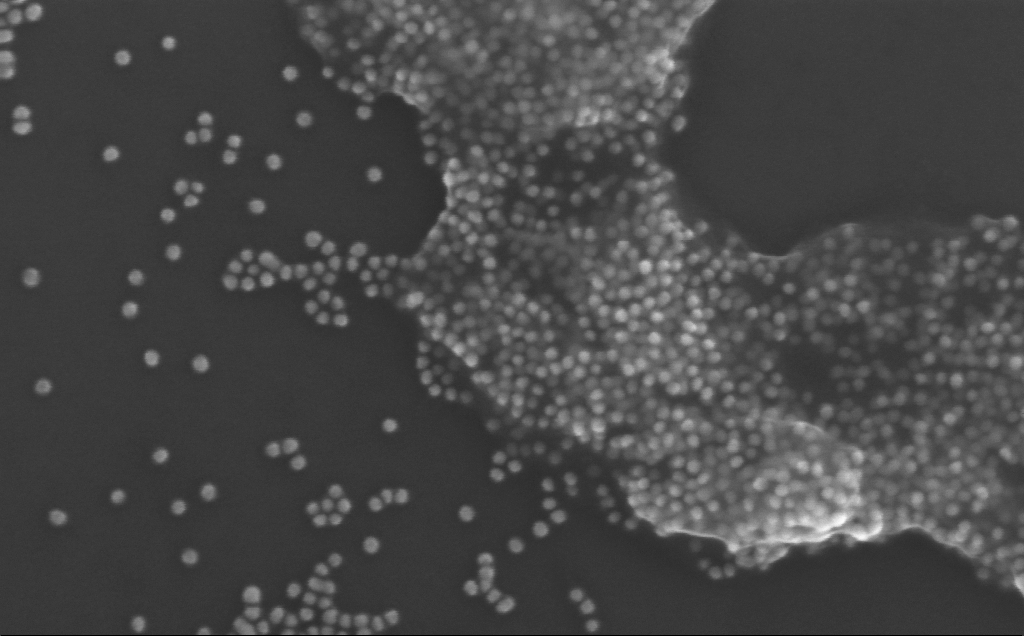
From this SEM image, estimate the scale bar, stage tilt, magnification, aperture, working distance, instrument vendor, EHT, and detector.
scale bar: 200 nm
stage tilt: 0°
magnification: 320.46 K X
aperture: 30 µm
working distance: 3 mm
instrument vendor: Zeiss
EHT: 10 kV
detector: InLens